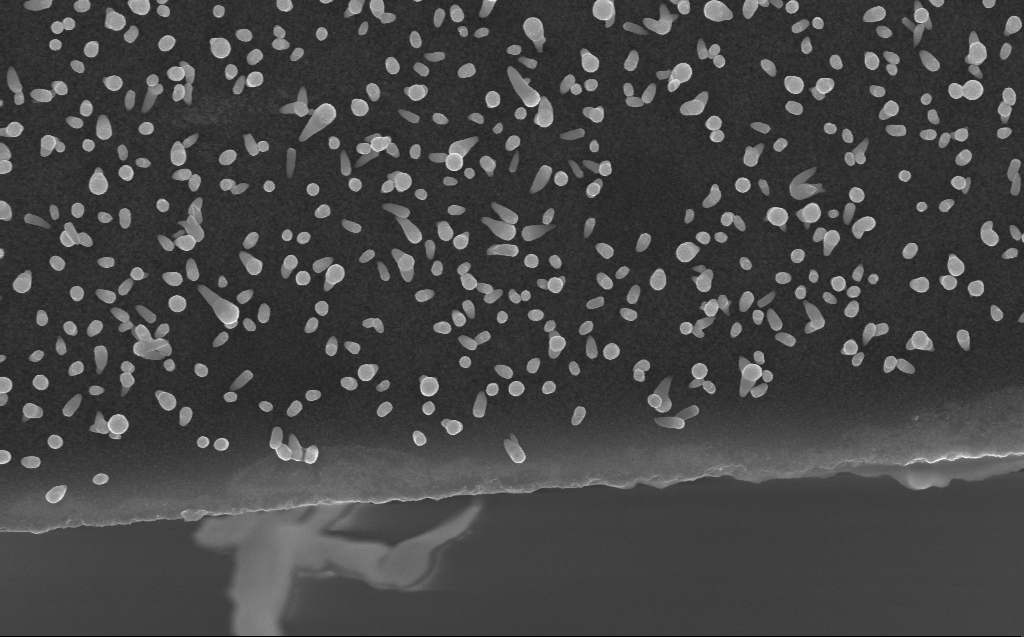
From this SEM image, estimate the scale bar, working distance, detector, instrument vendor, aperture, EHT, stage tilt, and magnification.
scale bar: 1000 nm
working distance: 3 mm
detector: InLens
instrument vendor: Zeiss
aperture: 30 µm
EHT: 10 kV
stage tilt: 0°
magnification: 38.56 K X